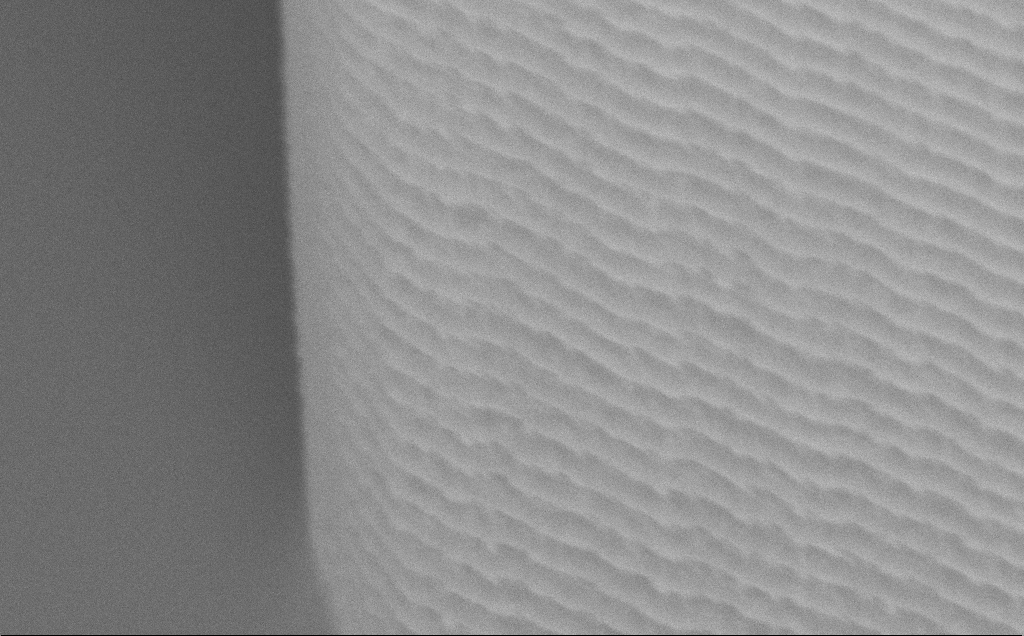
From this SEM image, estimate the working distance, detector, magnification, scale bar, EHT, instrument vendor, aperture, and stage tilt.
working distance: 7 mm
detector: InLens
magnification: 34.3 K X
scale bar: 2000 nm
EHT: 5 kV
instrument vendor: Zeiss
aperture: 30 µm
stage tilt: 45°